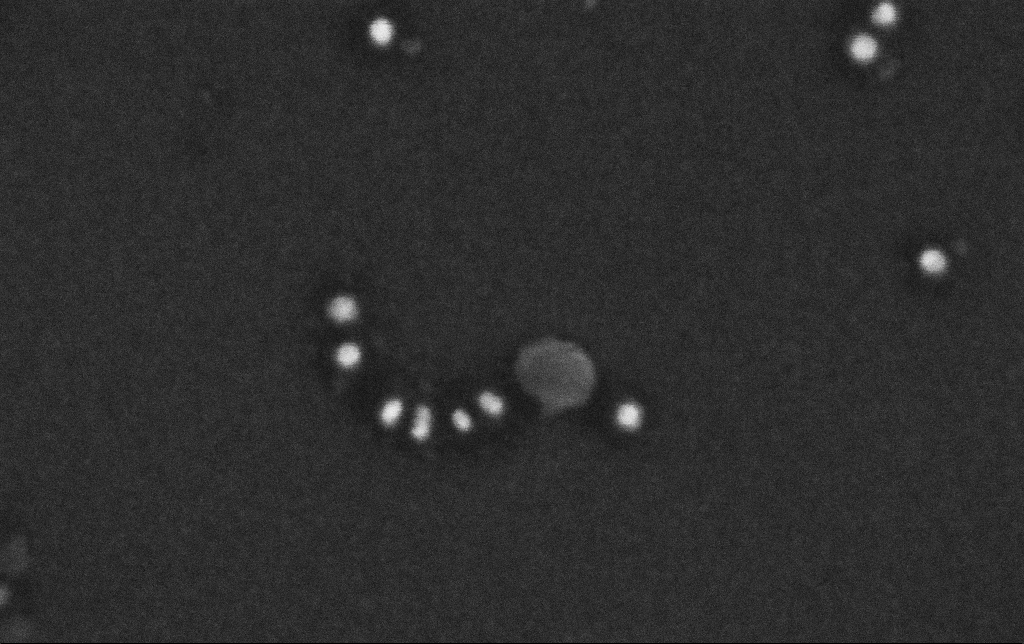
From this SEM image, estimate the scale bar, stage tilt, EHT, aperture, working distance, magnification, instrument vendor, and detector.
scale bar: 100 nm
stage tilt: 0°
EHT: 10 kV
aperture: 30 µm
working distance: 3.4 mm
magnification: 668.07 K X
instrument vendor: Zeiss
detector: InLens